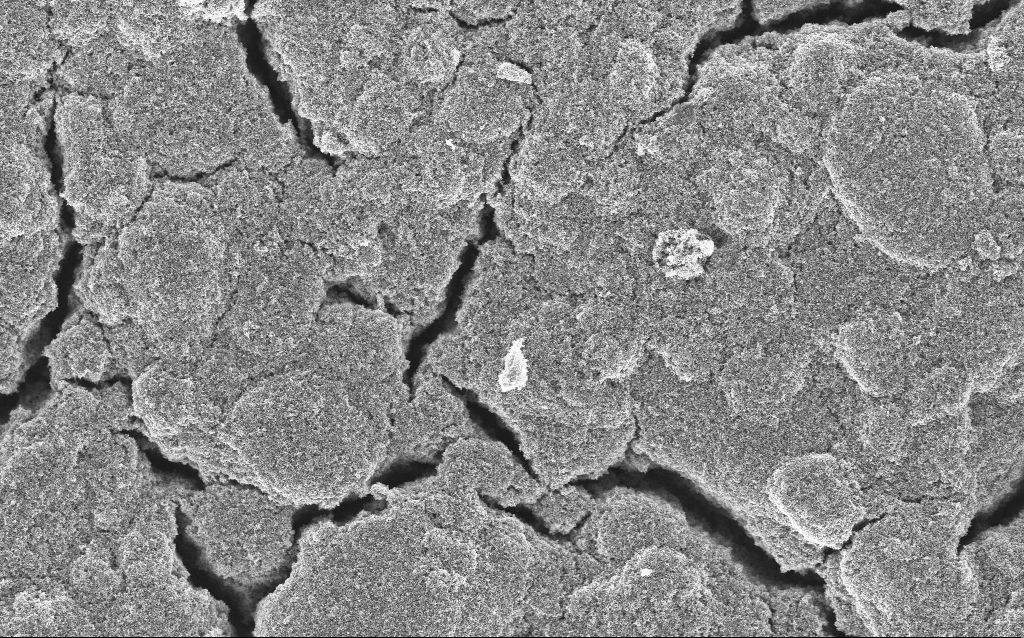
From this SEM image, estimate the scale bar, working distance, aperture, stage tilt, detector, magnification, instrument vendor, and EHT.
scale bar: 10000 nm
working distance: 4.2 mm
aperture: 30 µm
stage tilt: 0°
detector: InLens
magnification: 6.61 K X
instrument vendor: Zeiss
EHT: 5 kV